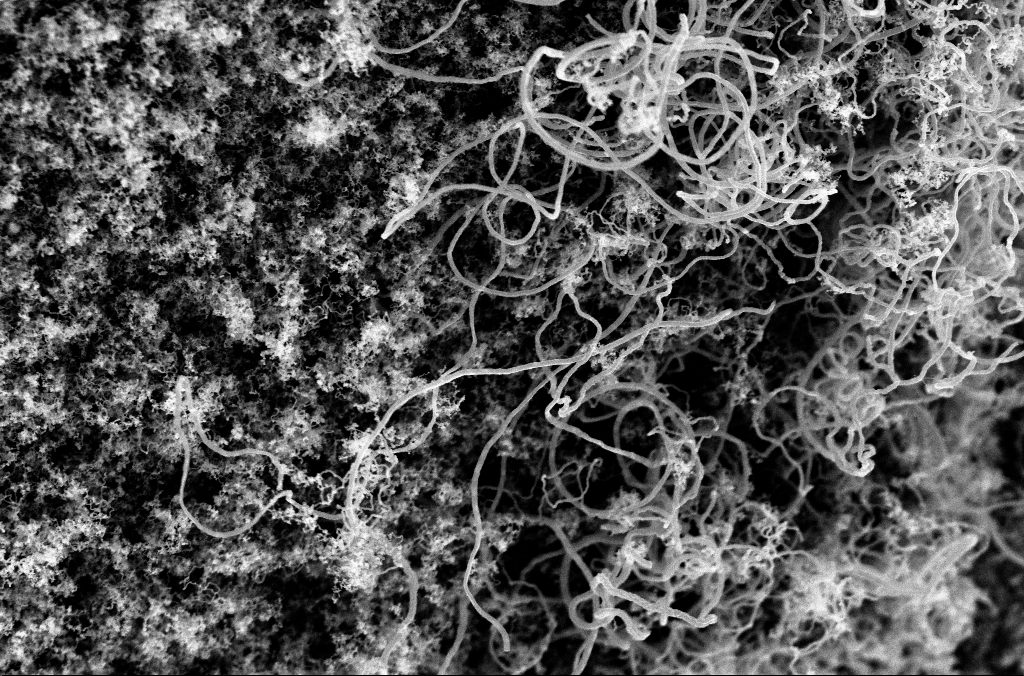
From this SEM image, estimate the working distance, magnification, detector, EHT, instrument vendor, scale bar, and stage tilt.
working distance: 5.1 mm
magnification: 25 K X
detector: InLens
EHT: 3 kV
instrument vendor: Zeiss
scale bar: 1000 nm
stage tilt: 0°